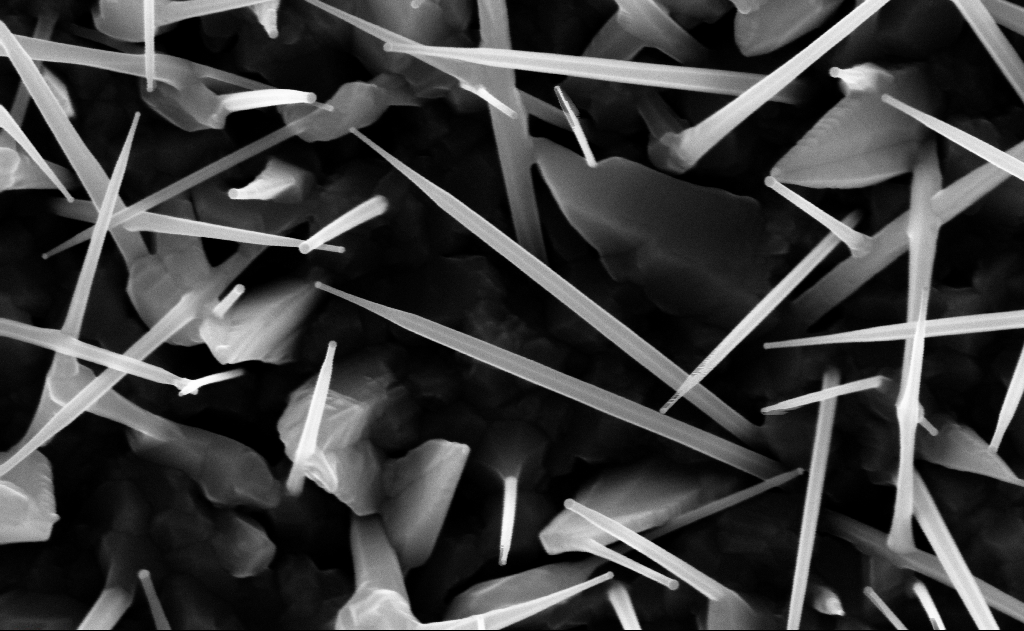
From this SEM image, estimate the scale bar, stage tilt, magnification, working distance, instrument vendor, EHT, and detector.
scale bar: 200 nm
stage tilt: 0°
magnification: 100 K X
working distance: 9 mm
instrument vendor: Zeiss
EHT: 10 kV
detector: InLens